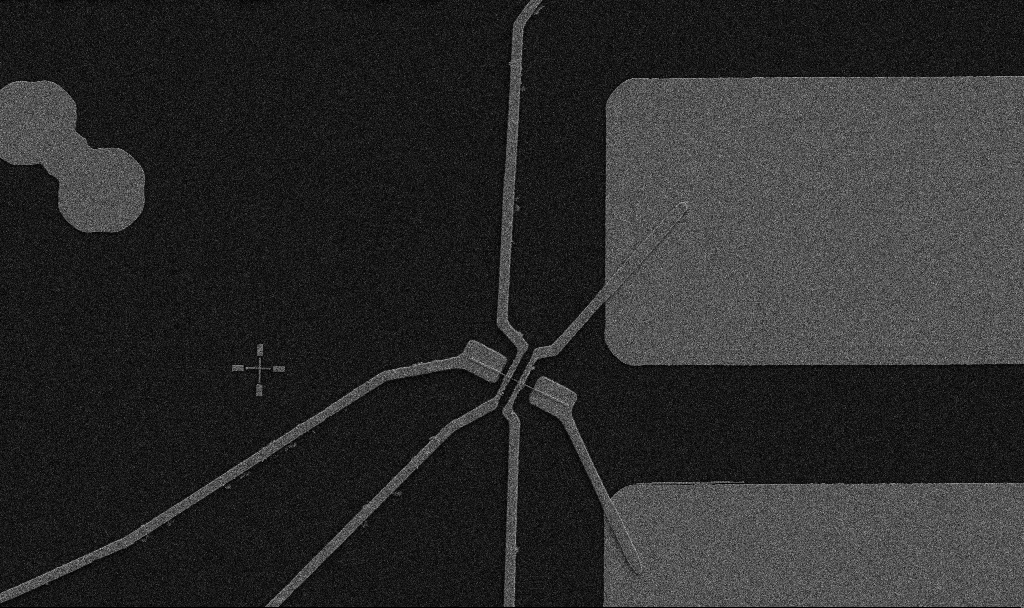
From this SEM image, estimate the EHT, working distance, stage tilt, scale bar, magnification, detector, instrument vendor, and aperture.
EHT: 5 kV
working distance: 10.7 mm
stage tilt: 0°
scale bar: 10000 nm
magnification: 5 K X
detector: SE2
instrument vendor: Zeiss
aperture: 30 µm